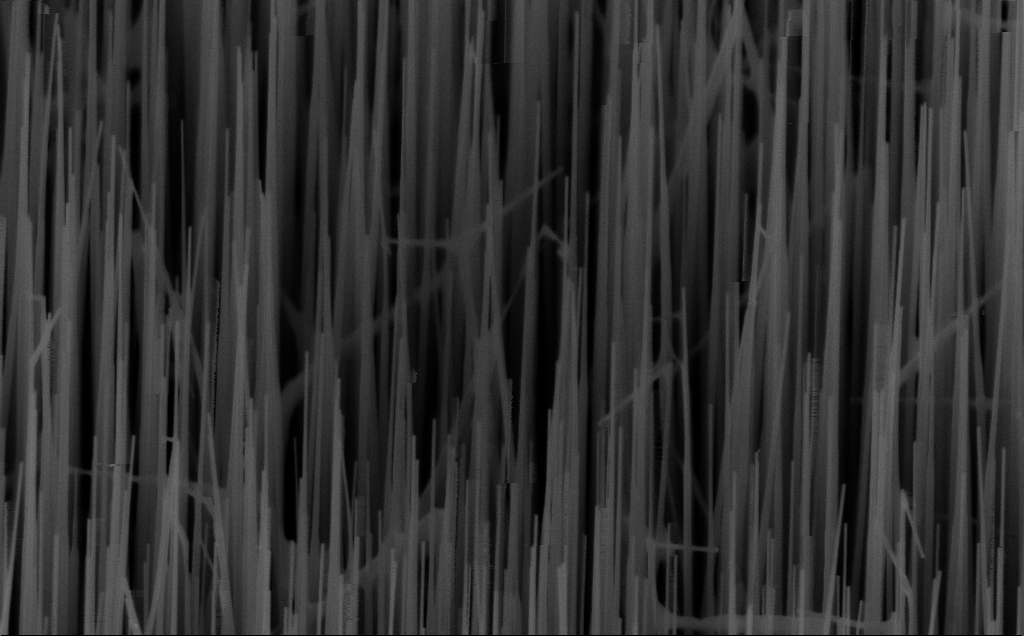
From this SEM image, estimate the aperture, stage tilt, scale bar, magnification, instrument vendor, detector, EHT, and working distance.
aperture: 30 µm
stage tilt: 45°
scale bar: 1000 nm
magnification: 40 K X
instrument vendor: Zeiss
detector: InLens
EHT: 10 kV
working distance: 6 mm